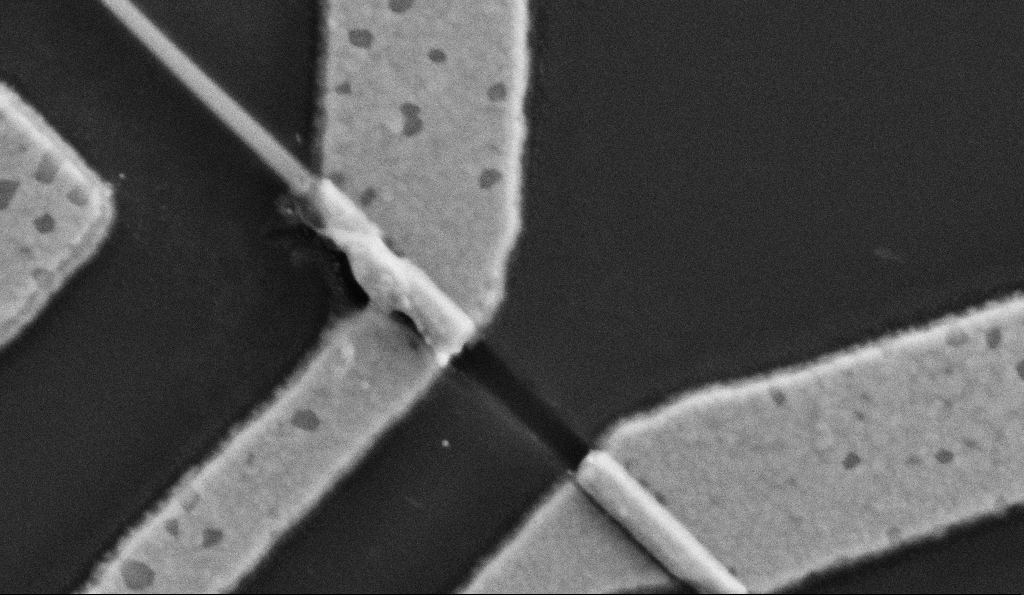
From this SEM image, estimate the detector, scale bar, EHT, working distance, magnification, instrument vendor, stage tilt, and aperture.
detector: SE2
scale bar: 200 nm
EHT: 5 kV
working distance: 7.7 mm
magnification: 100 K X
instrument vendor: Zeiss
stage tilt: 0°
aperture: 30 µm